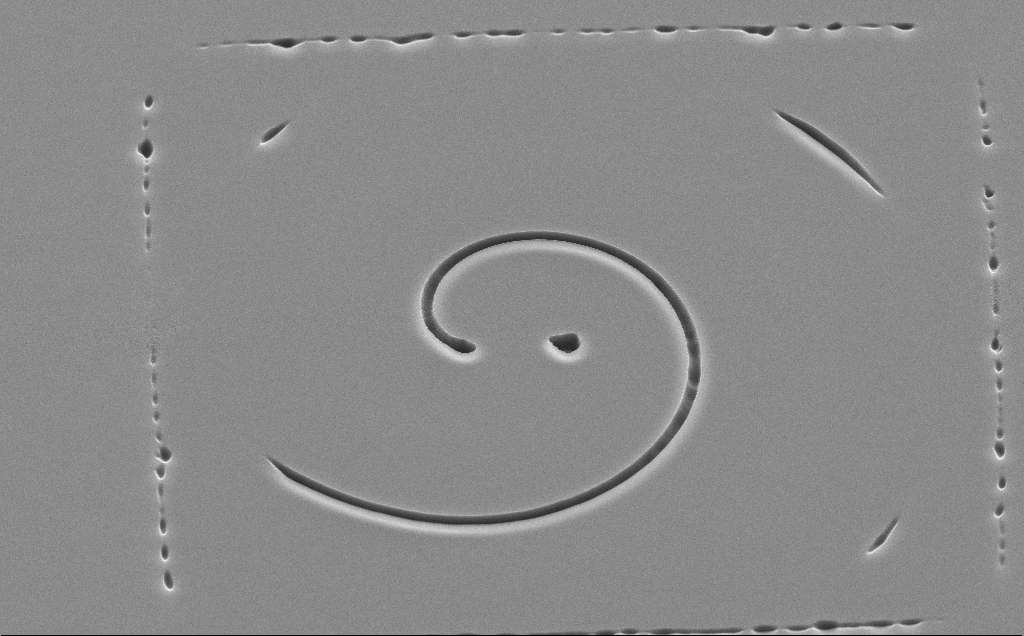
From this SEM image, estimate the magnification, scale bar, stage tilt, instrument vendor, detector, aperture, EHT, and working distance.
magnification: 3.64 K X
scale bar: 10000 nm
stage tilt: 45°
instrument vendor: Zeiss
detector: SE2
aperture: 30 µm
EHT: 10 kV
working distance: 11 mm